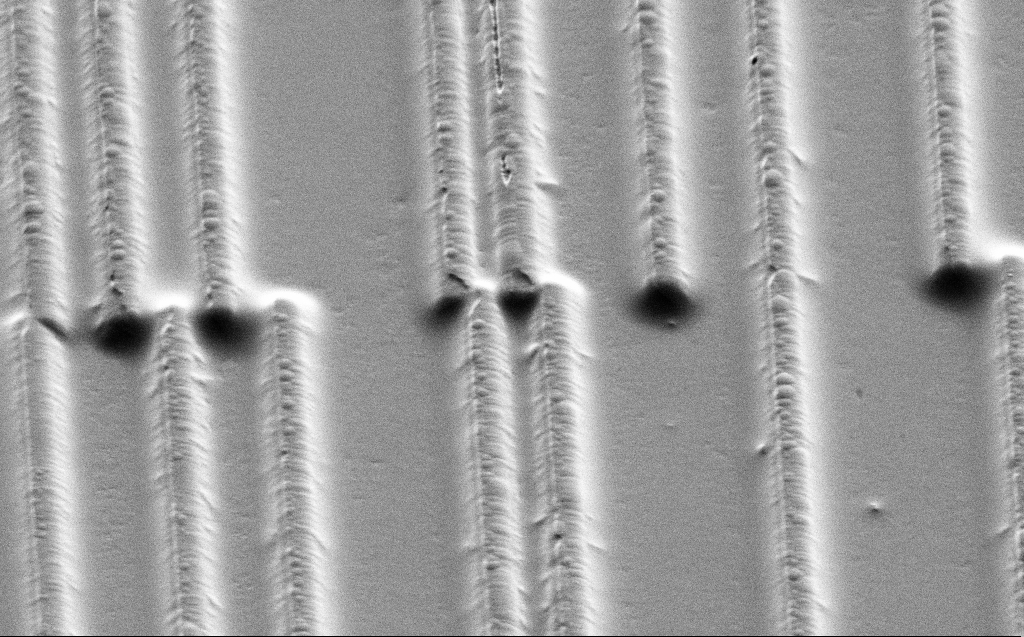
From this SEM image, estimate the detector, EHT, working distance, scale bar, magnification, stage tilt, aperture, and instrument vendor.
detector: SE2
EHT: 3 kV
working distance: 11 mm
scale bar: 10000 nm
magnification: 5.63 K X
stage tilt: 45°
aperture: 30 µm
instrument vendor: Zeiss